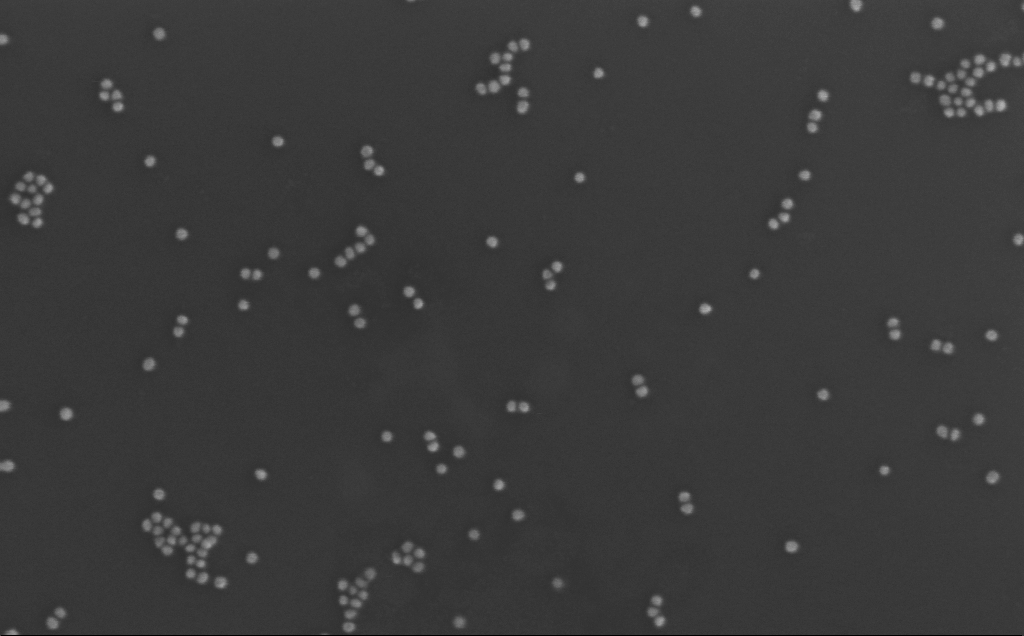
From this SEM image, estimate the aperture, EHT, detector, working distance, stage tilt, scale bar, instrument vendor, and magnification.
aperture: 30 µm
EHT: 10 kV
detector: InLens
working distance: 3 mm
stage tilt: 0°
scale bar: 200 nm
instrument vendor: Zeiss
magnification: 240.78 K X